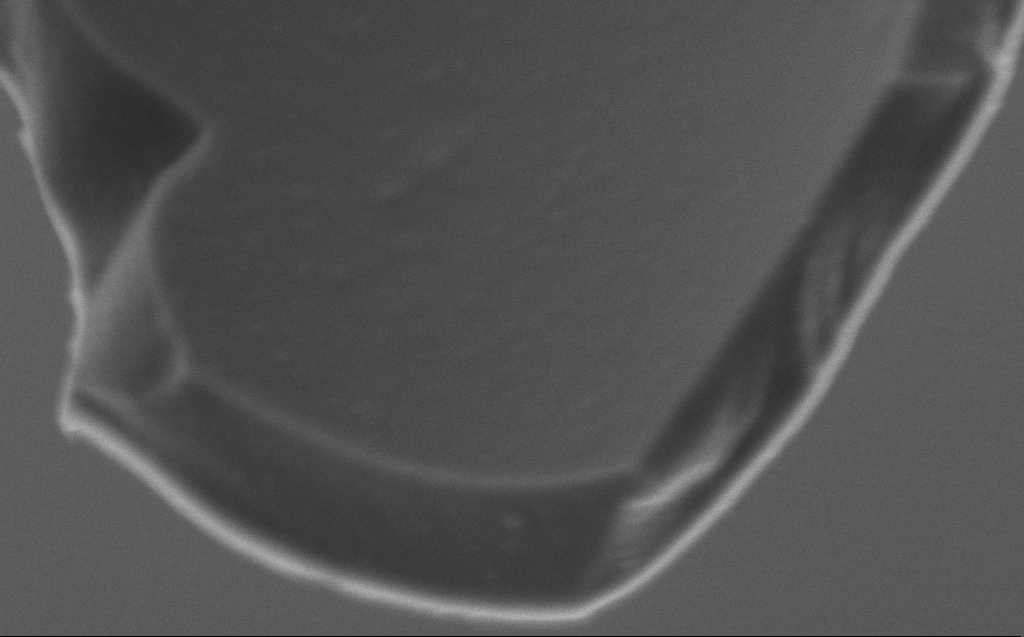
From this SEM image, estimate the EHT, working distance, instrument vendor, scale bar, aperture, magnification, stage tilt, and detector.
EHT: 2 kV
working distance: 5 mm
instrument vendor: Zeiss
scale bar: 200 nm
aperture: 30 µm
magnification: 150 K X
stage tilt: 45°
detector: SE2